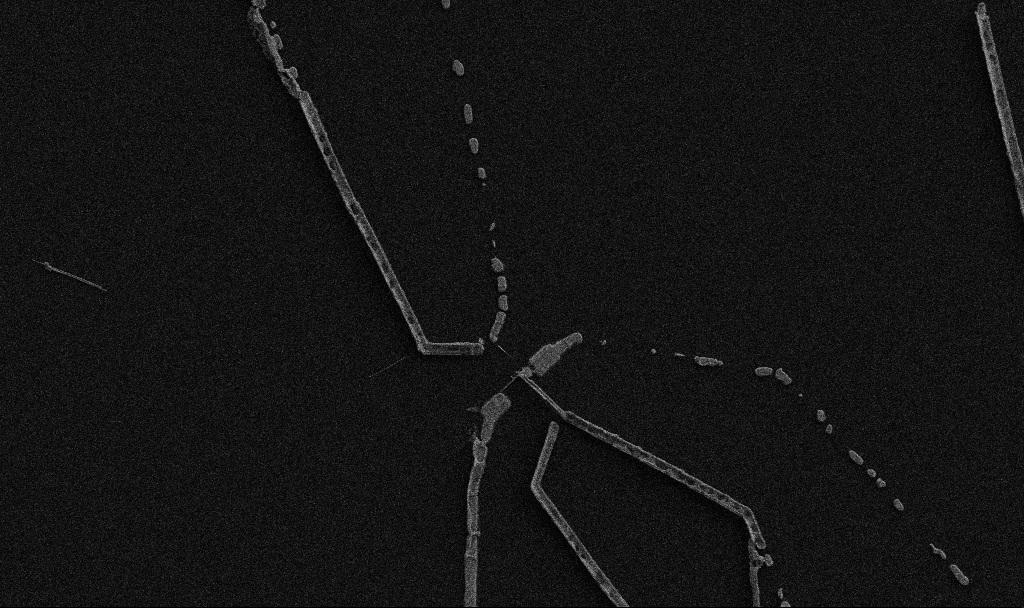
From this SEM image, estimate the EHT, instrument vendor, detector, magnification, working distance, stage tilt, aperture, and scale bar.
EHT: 5 kV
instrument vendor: Zeiss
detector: SE2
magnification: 5 K X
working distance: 10.7 mm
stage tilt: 0°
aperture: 30 µm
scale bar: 10000 nm